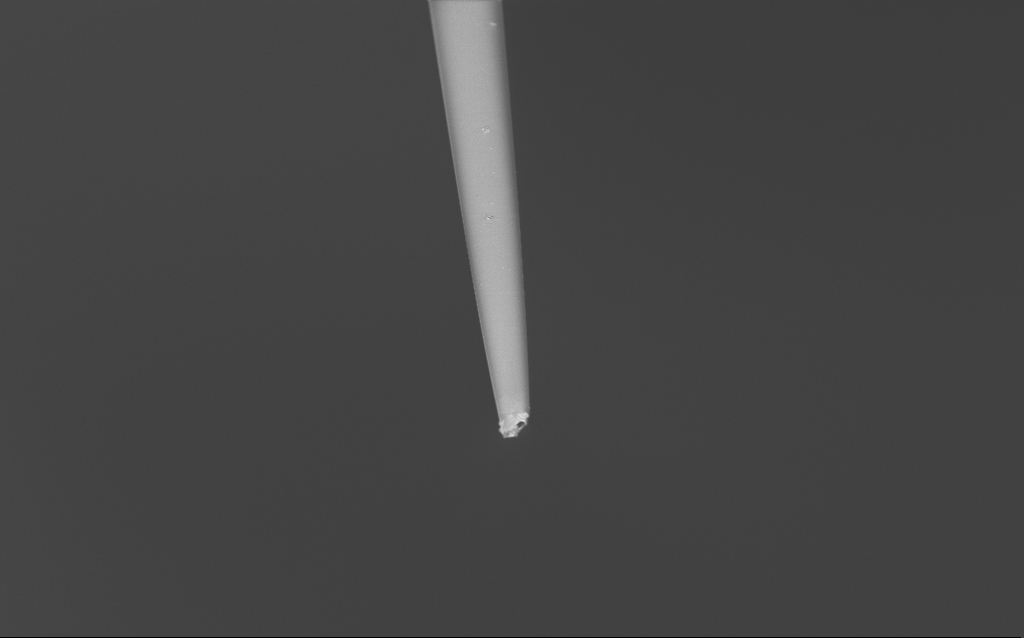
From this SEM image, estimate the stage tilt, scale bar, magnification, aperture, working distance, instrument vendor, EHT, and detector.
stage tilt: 45°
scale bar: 20000 nm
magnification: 1 K X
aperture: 30 µm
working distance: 6 mm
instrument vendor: Zeiss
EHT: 2 kV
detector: InLens